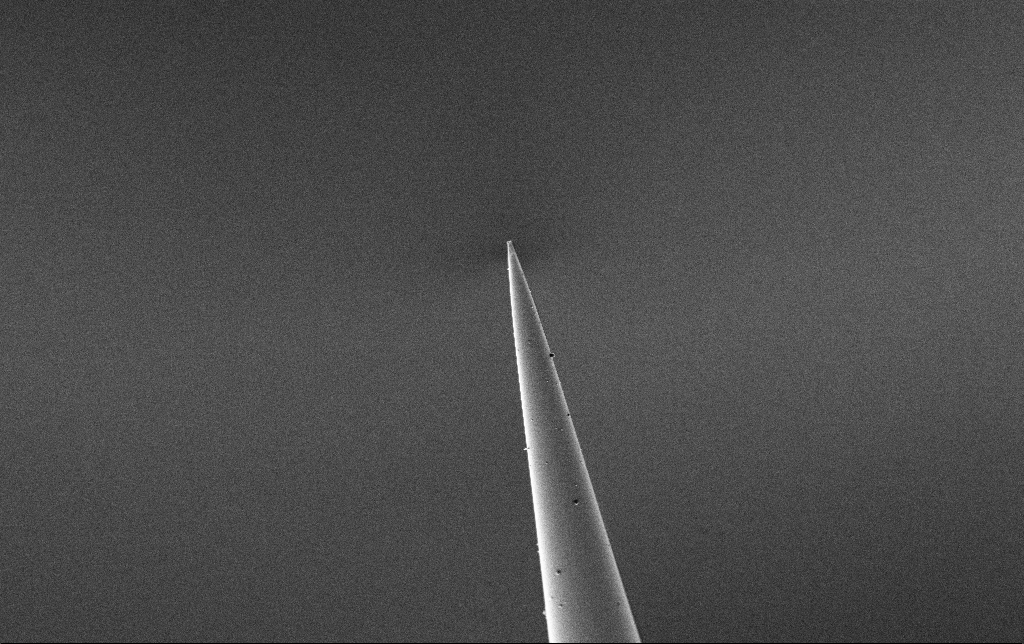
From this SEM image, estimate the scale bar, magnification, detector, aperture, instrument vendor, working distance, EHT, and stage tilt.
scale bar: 10000 nm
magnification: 5 K X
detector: SE2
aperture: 30 µm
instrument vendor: Zeiss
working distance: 7.6 mm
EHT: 2 kV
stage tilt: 45°